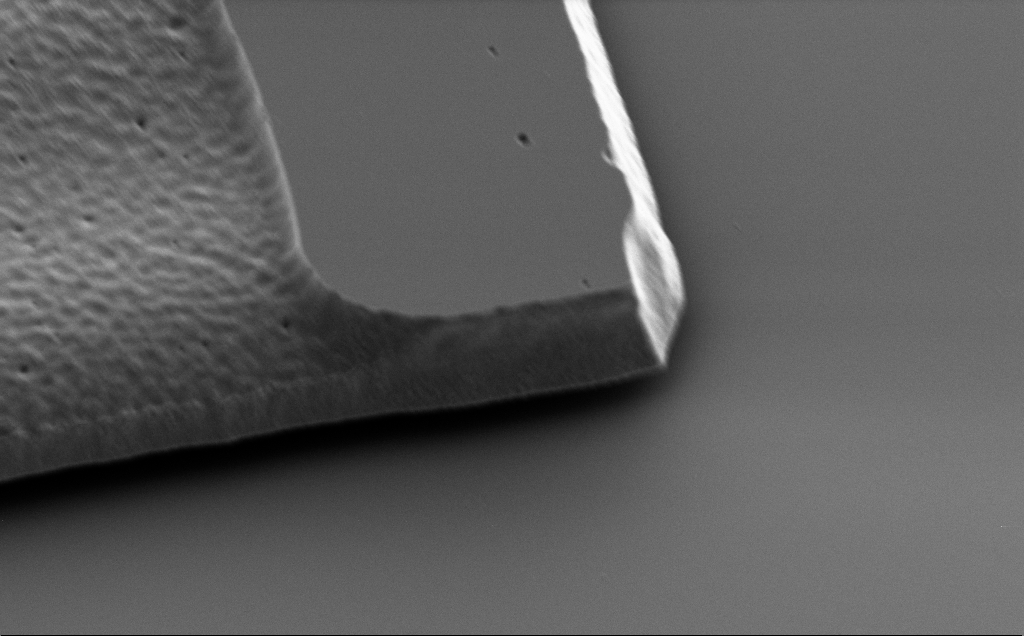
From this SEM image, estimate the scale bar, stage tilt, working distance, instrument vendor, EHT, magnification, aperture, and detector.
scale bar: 2000 nm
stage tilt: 43°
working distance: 10 mm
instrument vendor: Zeiss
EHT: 2 kV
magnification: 15.64 K X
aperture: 30 µm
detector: SE2